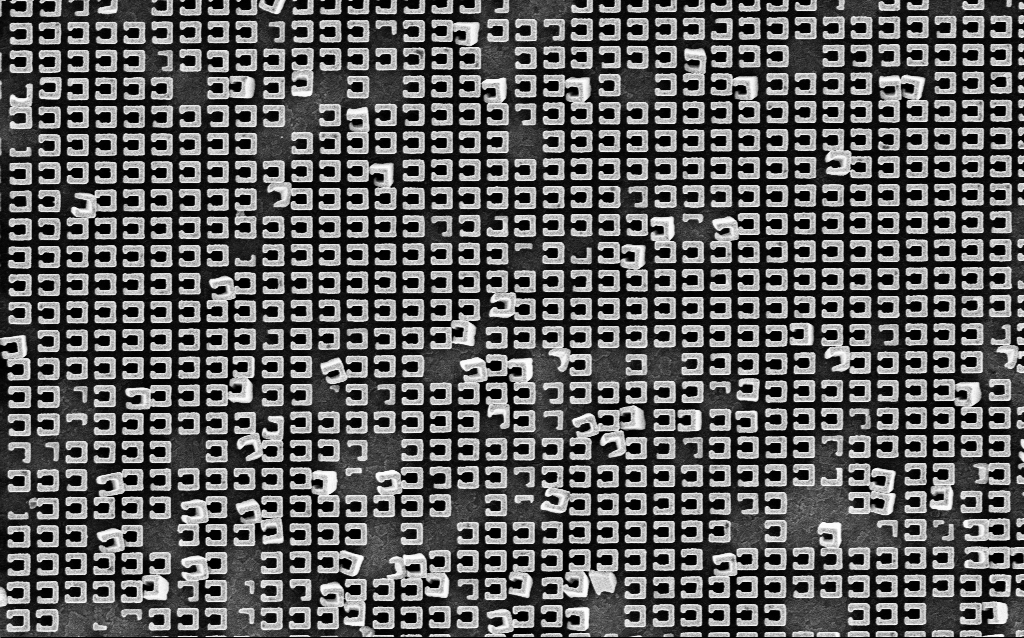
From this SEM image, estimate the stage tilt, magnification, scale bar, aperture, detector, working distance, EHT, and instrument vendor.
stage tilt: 0°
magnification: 22.33 K X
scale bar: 2000 nm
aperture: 30 µm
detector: InLens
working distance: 5.1 mm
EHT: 3 kV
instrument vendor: Zeiss